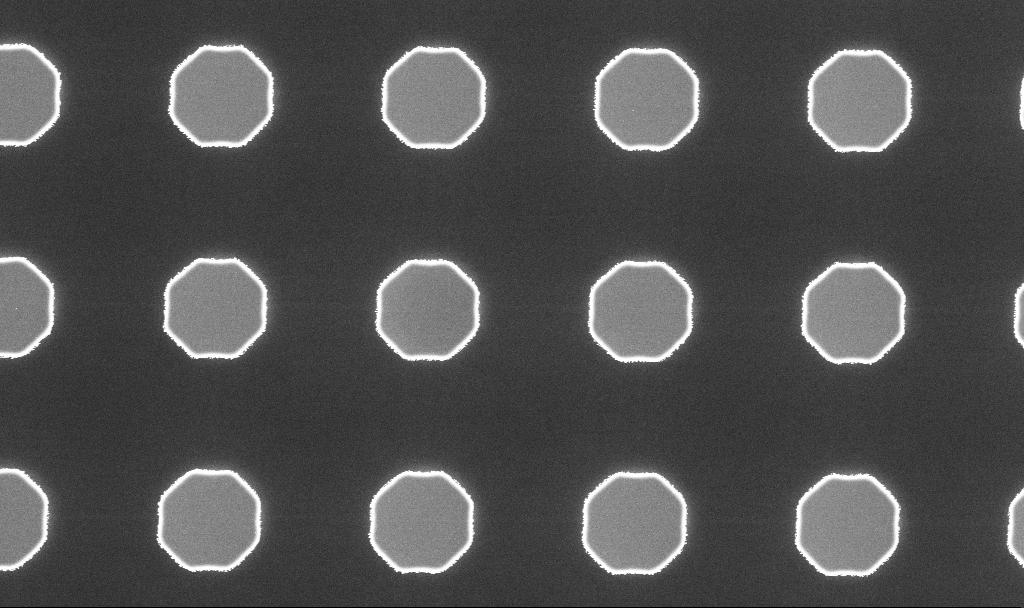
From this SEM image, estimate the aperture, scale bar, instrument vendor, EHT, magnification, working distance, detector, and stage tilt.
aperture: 30 µm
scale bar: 2000 nm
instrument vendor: Zeiss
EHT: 3 kV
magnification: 13.15 K X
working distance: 3.2 mm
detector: InLens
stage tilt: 0°